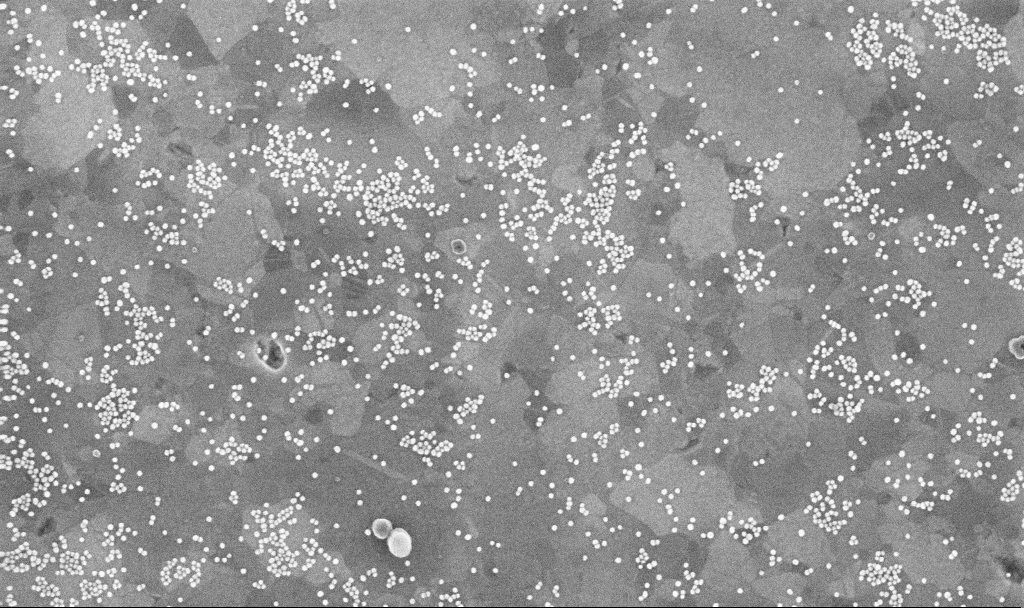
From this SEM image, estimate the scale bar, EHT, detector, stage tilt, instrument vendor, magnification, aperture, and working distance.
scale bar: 200 nm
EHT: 10 kV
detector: InLens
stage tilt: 0°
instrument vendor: Zeiss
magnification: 100 K X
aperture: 30 µm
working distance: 3.7 mm